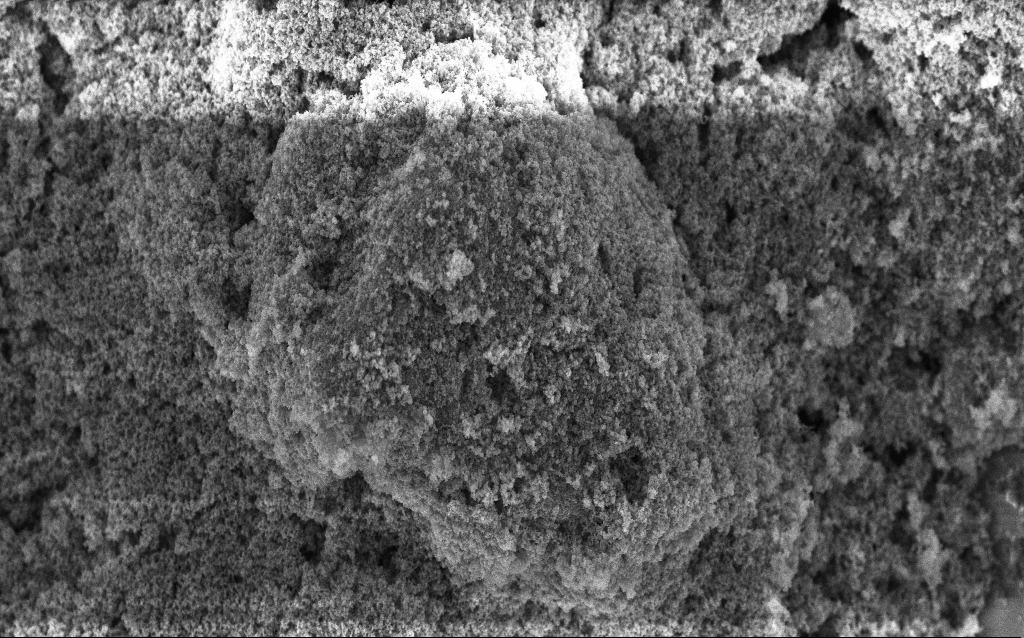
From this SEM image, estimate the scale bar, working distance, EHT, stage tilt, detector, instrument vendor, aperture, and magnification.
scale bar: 1000 nm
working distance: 2.5 mm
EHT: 5 kV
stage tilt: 0°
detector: InLens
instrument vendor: Zeiss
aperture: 30 µm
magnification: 20.87 K X